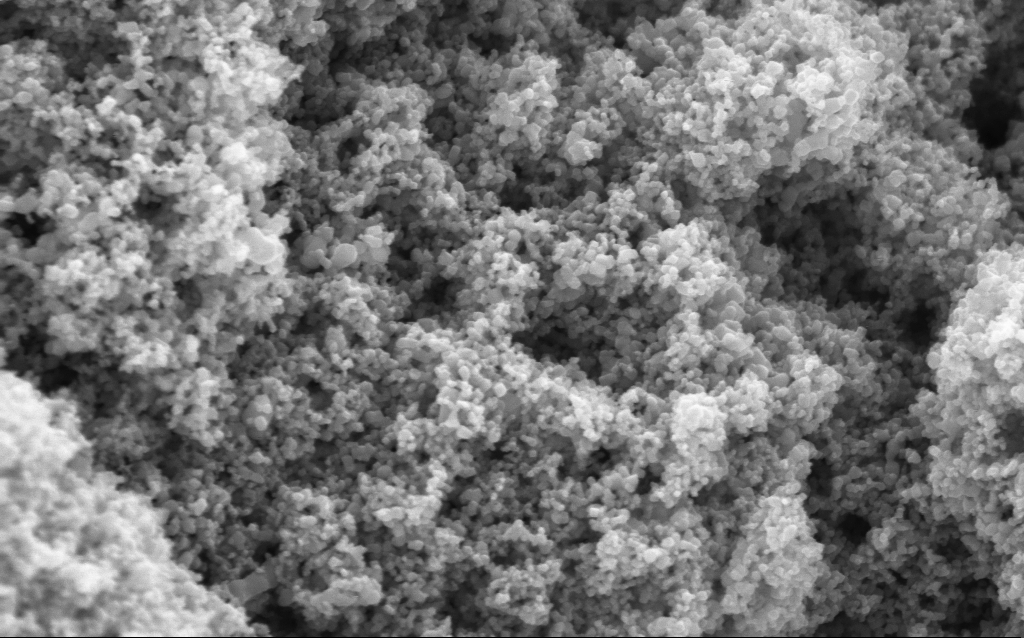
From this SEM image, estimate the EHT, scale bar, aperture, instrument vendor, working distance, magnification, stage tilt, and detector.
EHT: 5 kV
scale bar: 200 nm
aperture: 30 µm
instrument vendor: Zeiss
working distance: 4.5 mm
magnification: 114.65 K X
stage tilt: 0°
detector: InLens